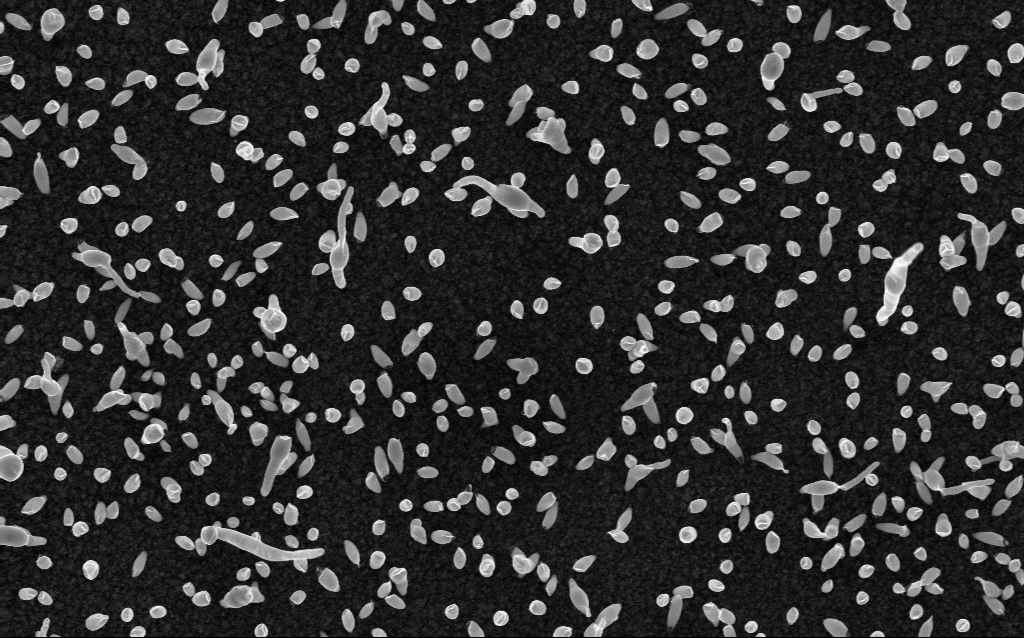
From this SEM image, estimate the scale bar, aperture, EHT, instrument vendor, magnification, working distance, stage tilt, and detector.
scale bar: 1000 nm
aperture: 30 µm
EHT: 5 kV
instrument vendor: Zeiss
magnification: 50 K X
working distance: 2.9 mm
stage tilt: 0°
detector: InLens